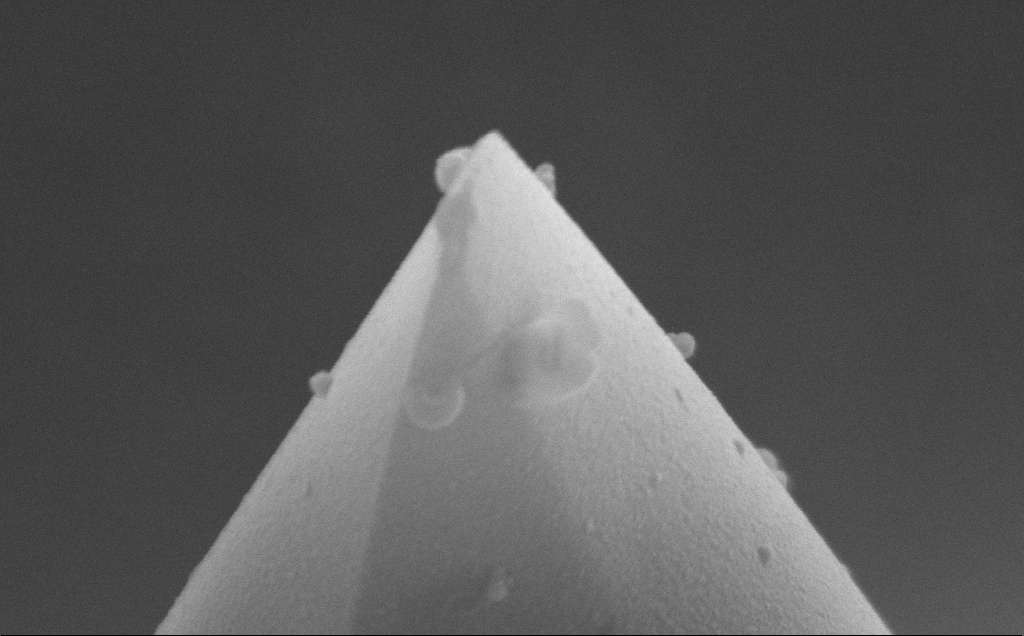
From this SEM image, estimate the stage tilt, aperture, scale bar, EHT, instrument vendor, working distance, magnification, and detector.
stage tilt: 30°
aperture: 30 µm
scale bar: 200 nm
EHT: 10 kV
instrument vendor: Zeiss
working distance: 9 mm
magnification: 189.91 K X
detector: SE2